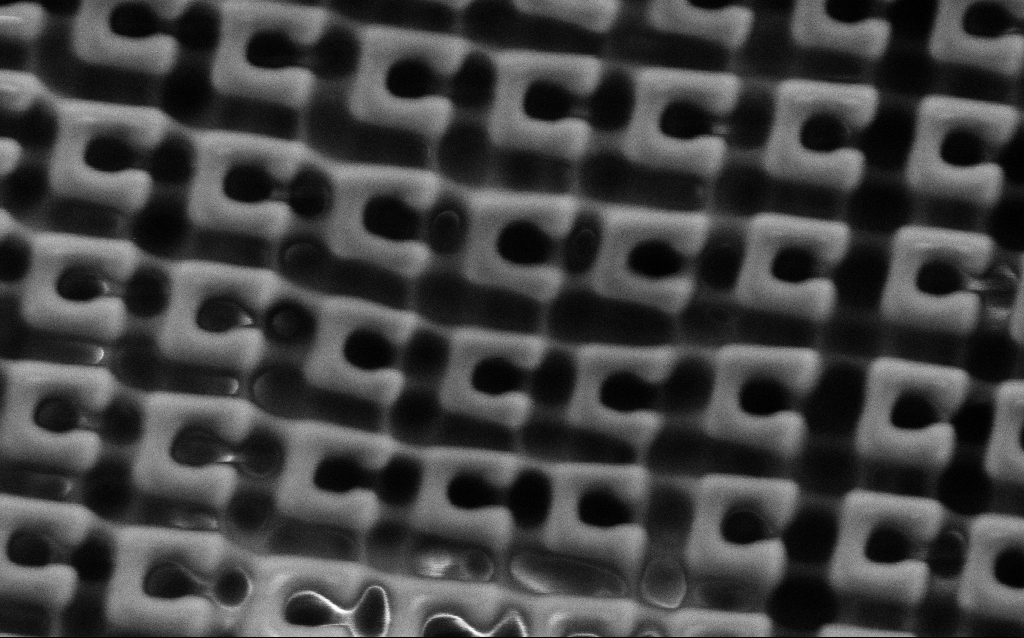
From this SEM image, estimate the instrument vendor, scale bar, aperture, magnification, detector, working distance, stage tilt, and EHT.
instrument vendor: Zeiss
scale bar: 200 nm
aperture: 30 µm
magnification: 109.83 K X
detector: SE2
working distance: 6.2 mm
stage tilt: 0°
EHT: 1.5 kV